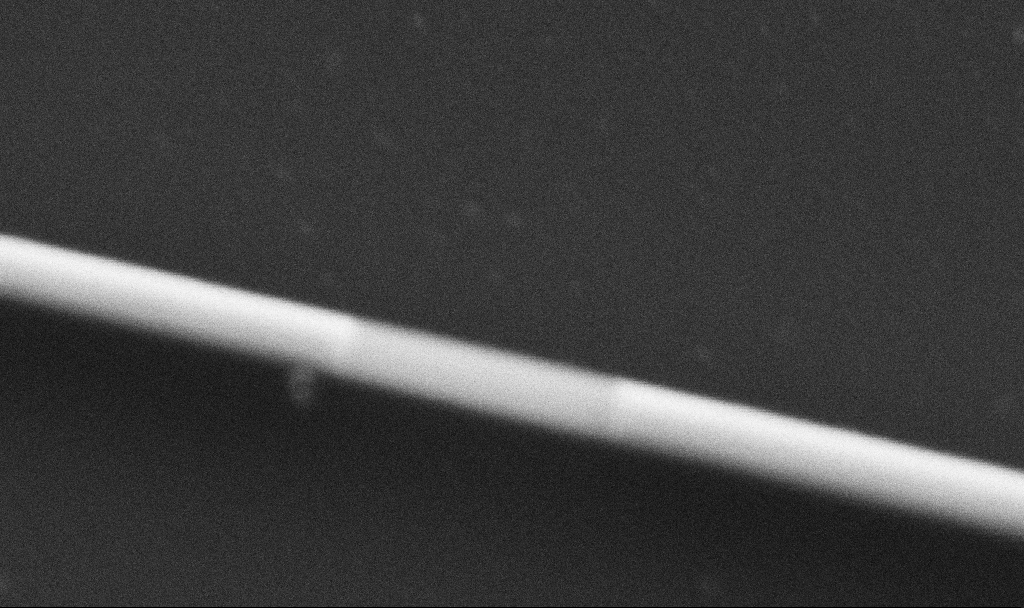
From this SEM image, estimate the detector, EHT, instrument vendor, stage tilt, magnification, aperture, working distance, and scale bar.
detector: SE2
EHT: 5 kV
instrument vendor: Zeiss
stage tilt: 0°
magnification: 300 K X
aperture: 30 µm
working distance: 8.7 mm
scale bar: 100 nm